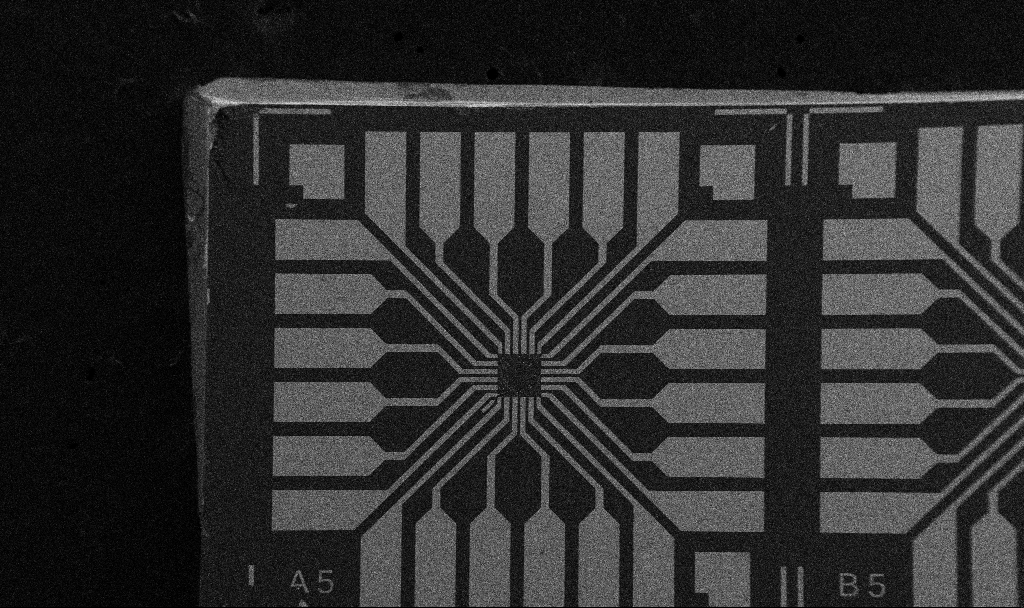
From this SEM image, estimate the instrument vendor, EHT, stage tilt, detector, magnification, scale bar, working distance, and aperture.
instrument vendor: Zeiss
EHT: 5 kV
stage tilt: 0°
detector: SE2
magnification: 0.1 K X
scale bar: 200000 nm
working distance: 10.7 mm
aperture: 30 µm